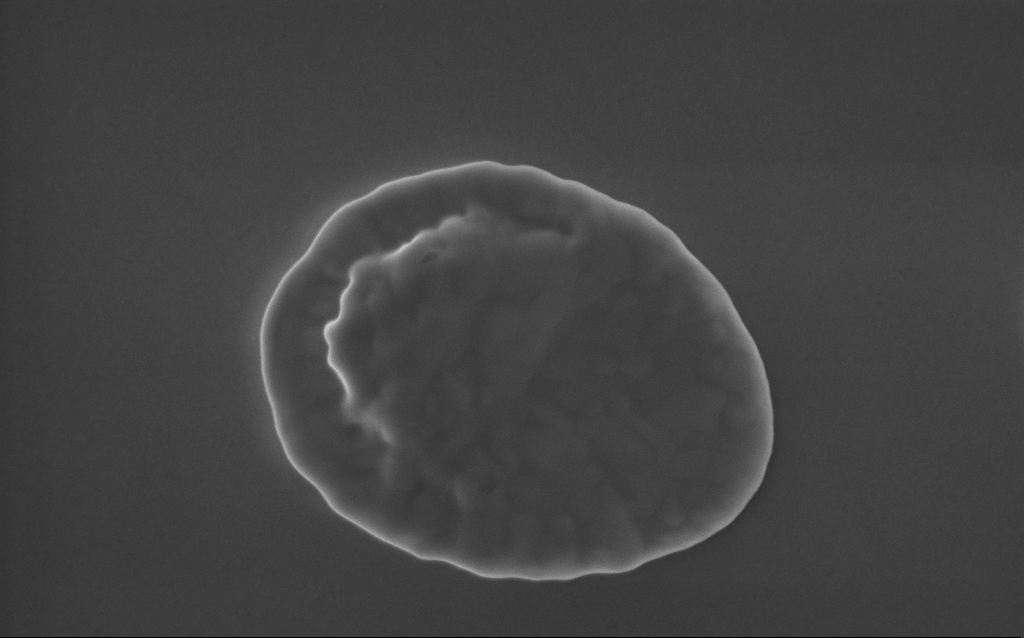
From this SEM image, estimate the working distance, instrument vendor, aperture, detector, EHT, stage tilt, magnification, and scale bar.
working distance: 3 mm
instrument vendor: Zeiss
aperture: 30 µm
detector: InLens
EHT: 5 kV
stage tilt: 0°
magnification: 92.92 K X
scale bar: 200 nm